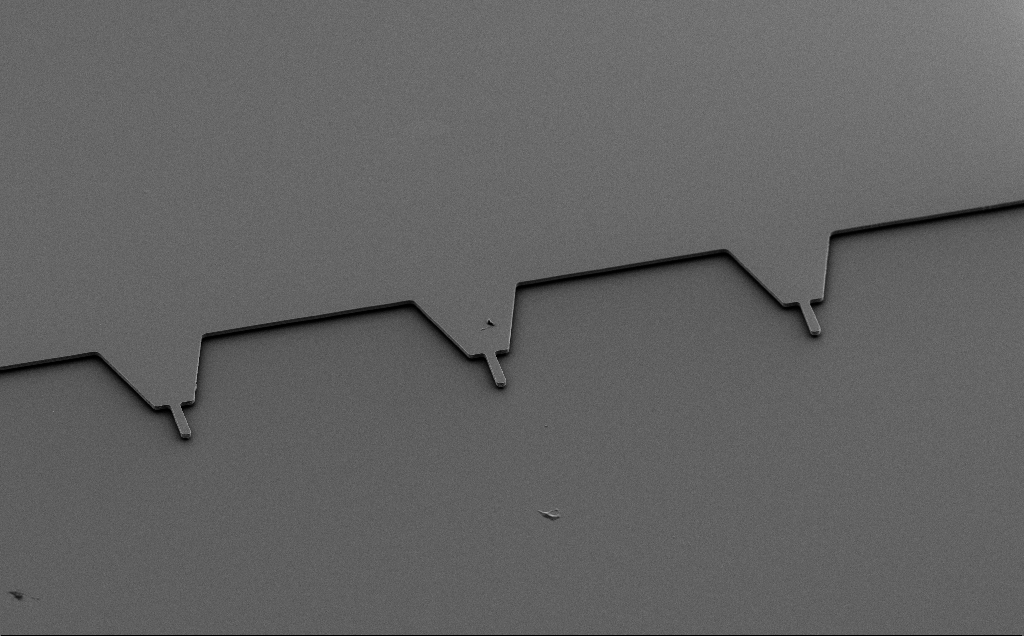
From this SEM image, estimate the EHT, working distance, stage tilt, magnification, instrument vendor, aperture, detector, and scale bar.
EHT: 5 kV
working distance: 10 mm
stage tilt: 50°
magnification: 0.744 K X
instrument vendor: Zeiss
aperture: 30 µm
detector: SE2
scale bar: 20000 nm